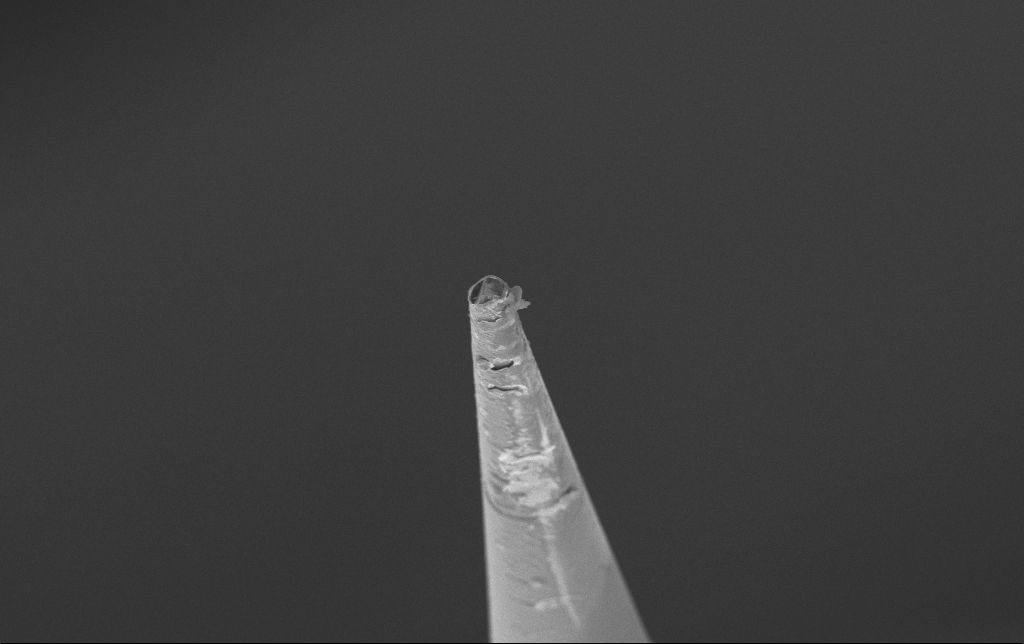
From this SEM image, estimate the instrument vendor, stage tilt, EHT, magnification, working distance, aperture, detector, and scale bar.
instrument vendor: Zeiss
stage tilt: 69.3°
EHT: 10 kV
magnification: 3.11 K X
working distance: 9.9 mm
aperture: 30 µm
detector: SE2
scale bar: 20000 nm